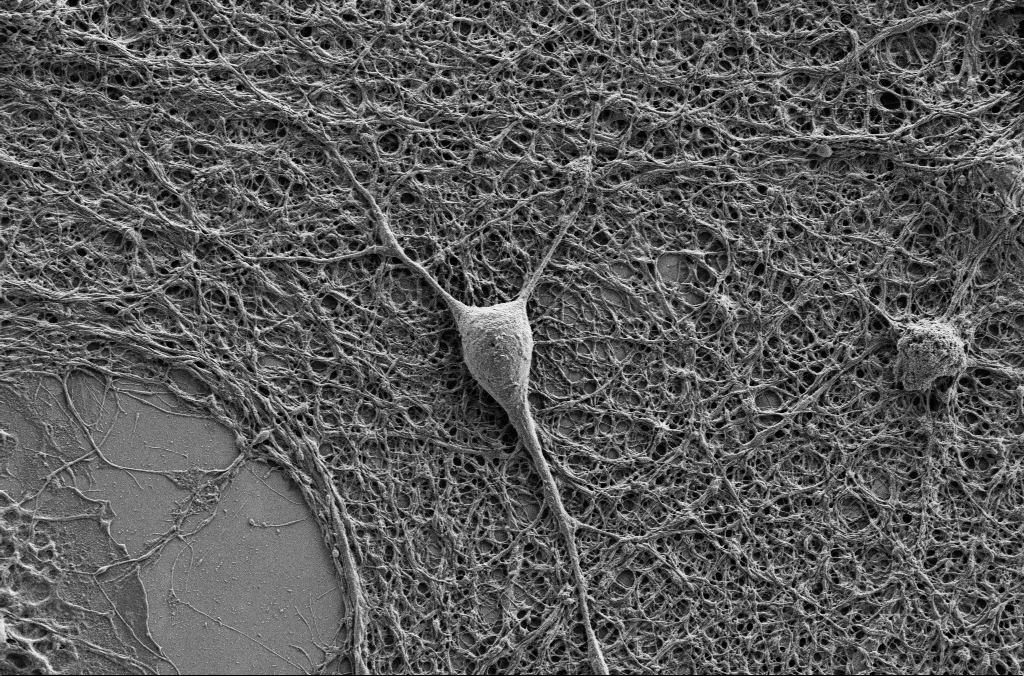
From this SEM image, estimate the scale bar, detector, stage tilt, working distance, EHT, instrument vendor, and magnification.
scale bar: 10000 nm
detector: SE2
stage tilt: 0°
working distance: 4.1 mm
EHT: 1 kV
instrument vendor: Zeiss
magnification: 5 K X